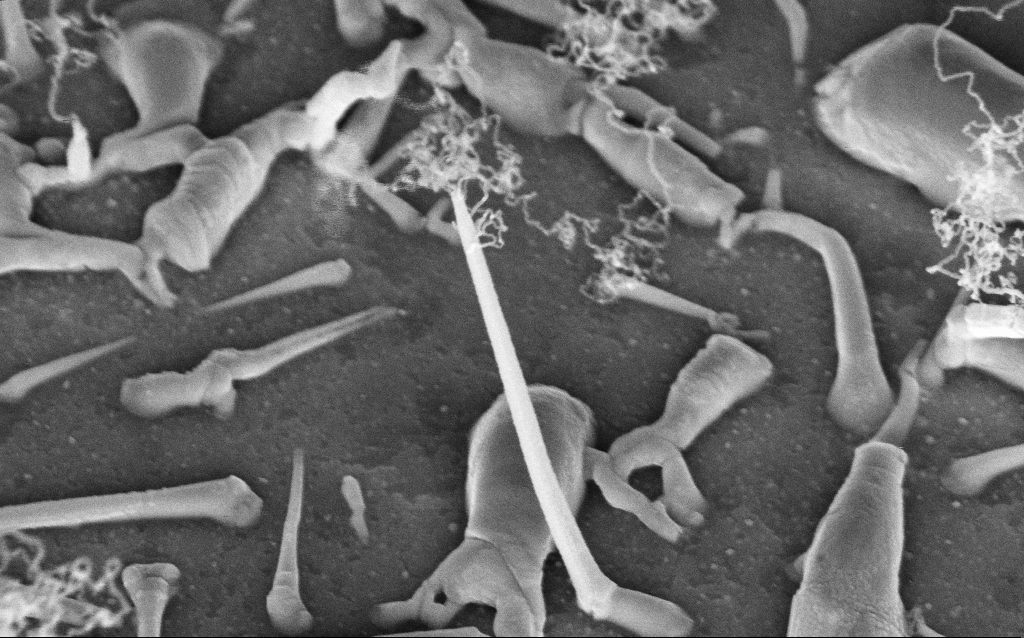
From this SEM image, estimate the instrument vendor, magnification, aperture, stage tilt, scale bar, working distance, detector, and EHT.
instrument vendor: Zeiss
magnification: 79.72 K X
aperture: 30 µm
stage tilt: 42°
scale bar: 200 nm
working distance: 8 mm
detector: InLens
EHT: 5 kV